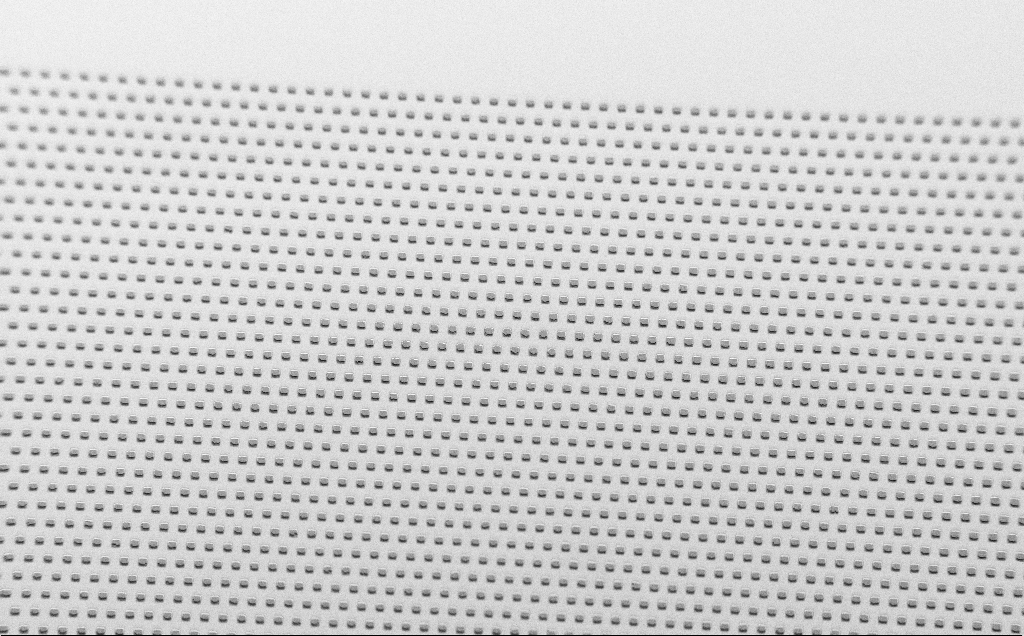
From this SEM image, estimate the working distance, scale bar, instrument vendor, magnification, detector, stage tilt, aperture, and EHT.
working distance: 7 mm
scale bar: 200000 nm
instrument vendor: Zeiss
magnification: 0.335 K X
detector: SE2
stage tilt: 45°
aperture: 30 µm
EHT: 1.5 kV